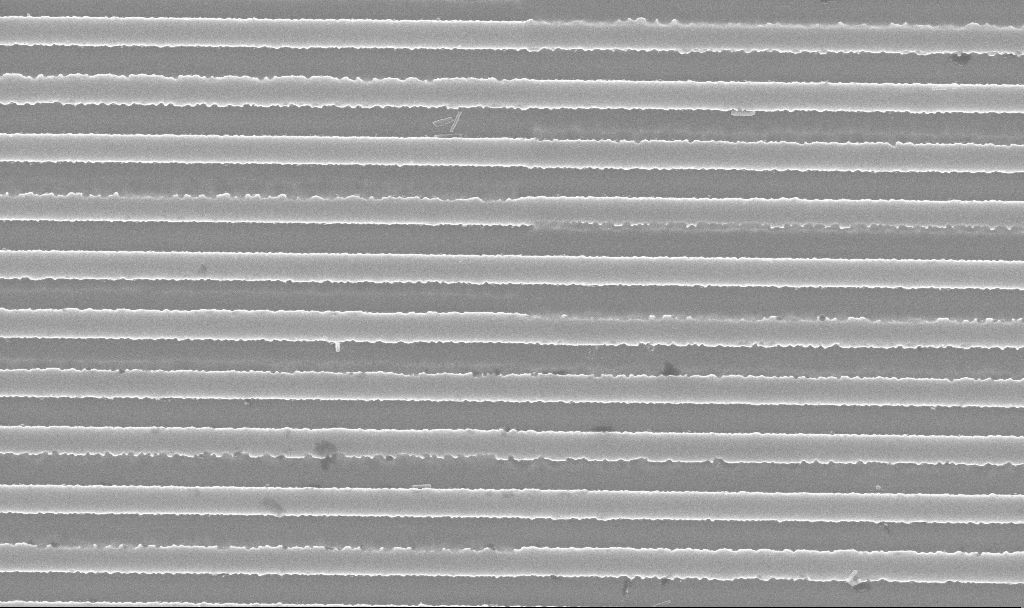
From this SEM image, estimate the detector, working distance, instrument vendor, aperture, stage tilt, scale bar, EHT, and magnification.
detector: InLens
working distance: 9.9 mm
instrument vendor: Zeiss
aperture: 30 µm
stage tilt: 0°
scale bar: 2000 nm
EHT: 5 kV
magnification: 32.77 K X